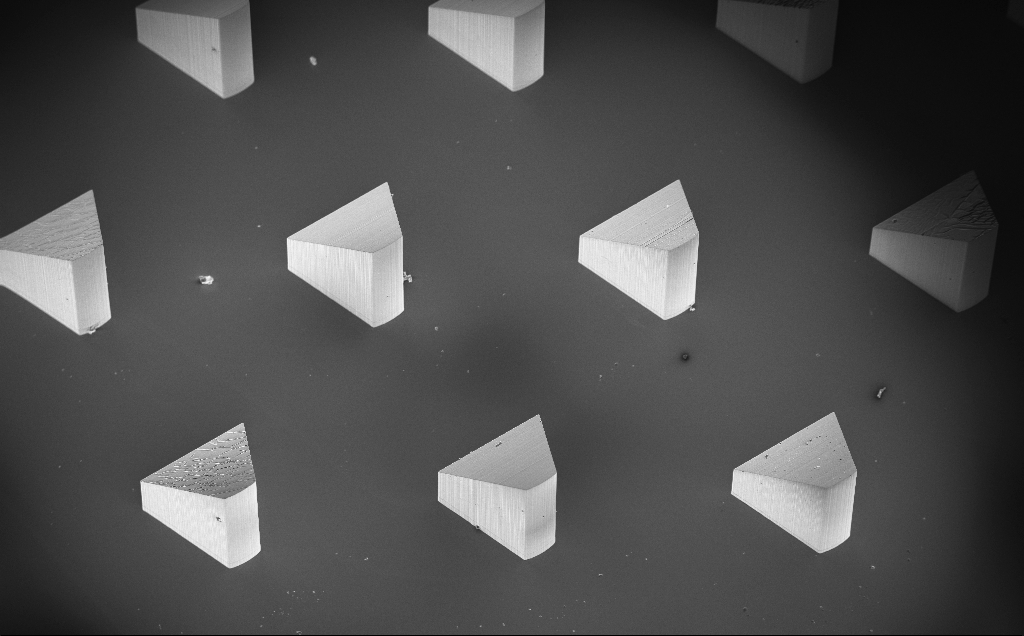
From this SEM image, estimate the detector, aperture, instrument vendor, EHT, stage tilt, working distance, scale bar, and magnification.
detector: InLens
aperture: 30 µm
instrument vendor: Zeiss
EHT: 10 kV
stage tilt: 20°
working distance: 9 mm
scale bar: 200000 nm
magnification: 0.111 K X